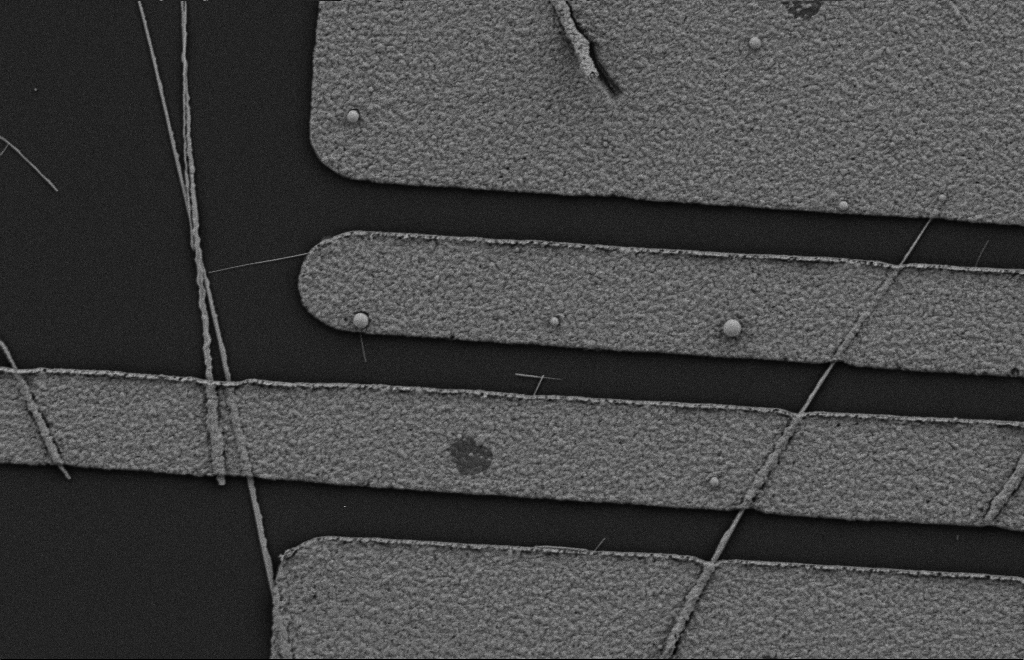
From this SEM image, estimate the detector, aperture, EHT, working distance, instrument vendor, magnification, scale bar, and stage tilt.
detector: SE2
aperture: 20 µm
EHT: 2 kV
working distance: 10 mm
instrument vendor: Zeiss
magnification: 14 K X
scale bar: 2000 nm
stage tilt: -0.3°